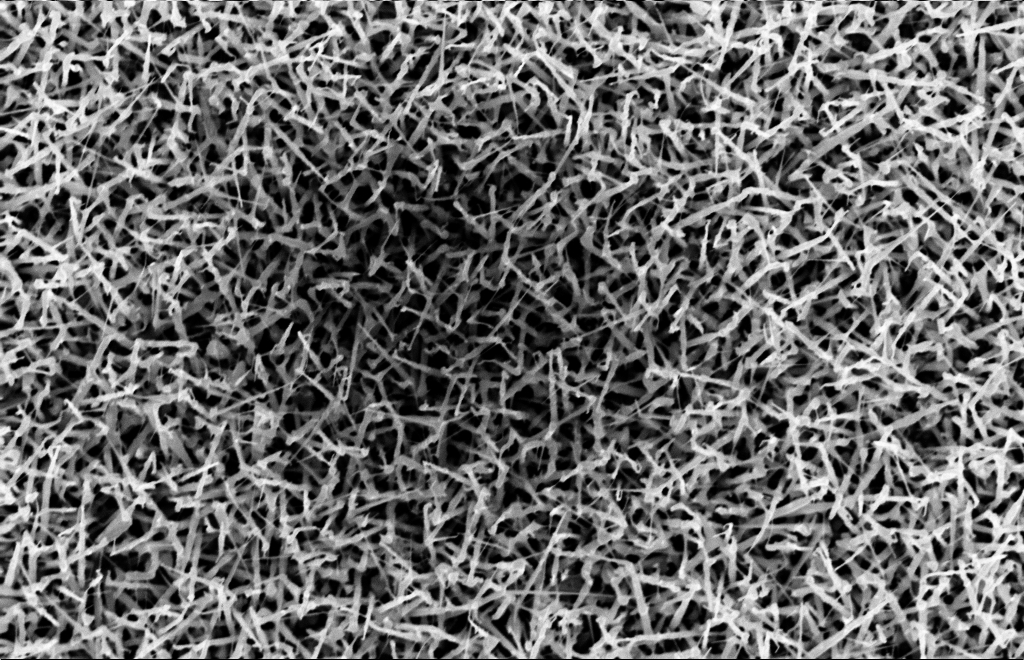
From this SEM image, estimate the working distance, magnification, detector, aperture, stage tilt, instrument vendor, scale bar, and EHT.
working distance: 15 mm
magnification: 20 K X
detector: InLens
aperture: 30 µm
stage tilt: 0°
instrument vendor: Zeiss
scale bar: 2000 nm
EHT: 10 kV